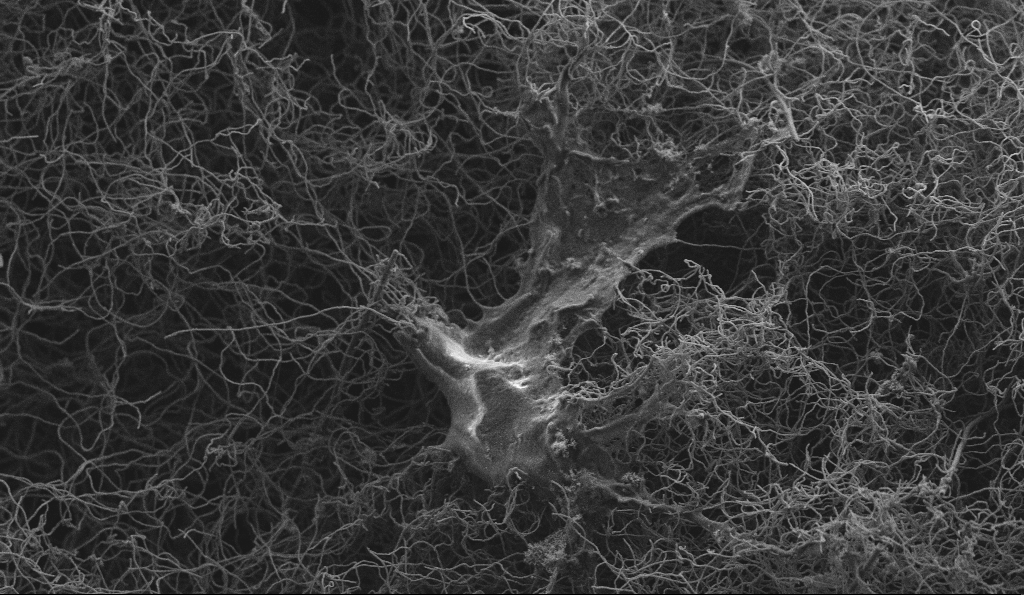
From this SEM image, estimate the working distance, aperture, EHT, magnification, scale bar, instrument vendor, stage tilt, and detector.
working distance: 4.6 mm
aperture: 30 µm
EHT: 3 kV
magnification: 5 K X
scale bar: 10000 nm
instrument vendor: Zeiss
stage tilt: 0°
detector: SE2